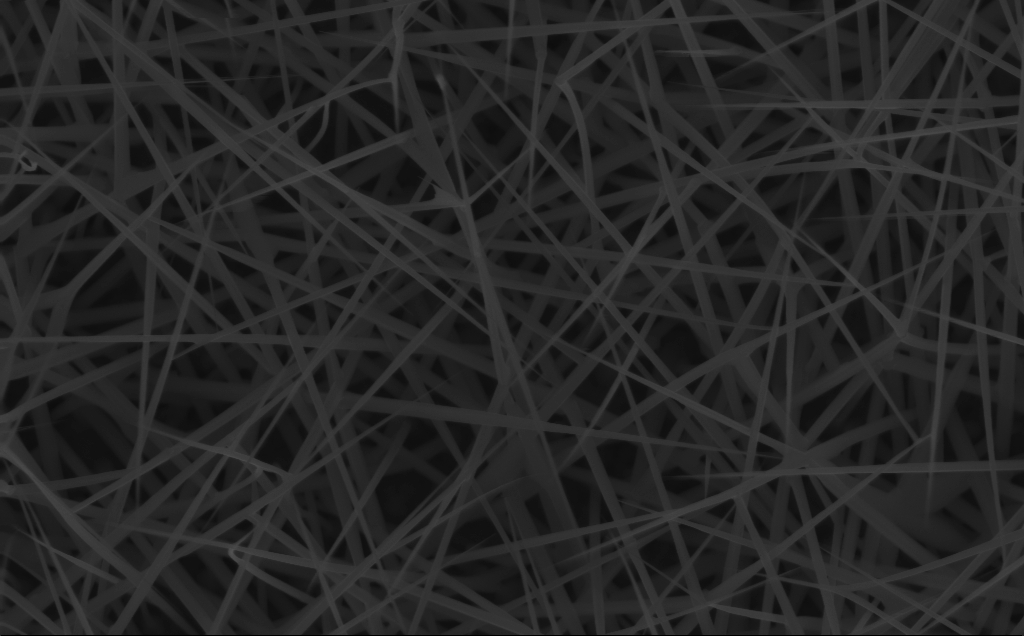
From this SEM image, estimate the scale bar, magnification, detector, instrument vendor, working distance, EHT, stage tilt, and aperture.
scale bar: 1000 nm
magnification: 40 K X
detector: InLens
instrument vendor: Zeiss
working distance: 4 mm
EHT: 10 kV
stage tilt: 0°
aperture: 30 µm